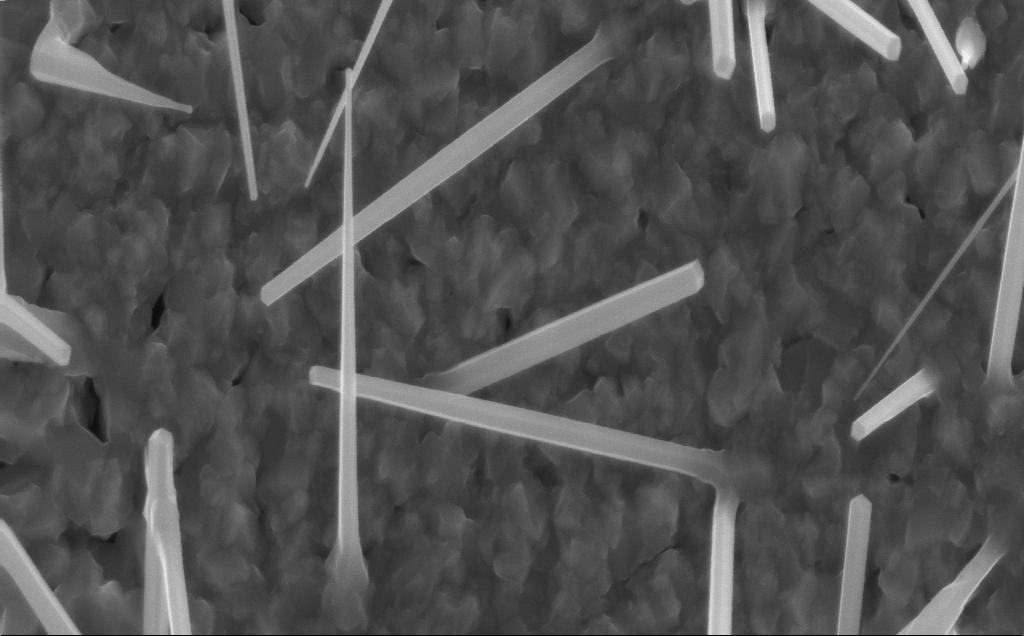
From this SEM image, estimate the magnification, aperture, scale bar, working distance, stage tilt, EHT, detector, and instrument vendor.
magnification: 40 K X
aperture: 30 µm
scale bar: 1000 nm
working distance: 4 mm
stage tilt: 0°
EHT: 10 kV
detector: InLens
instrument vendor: Zeiss